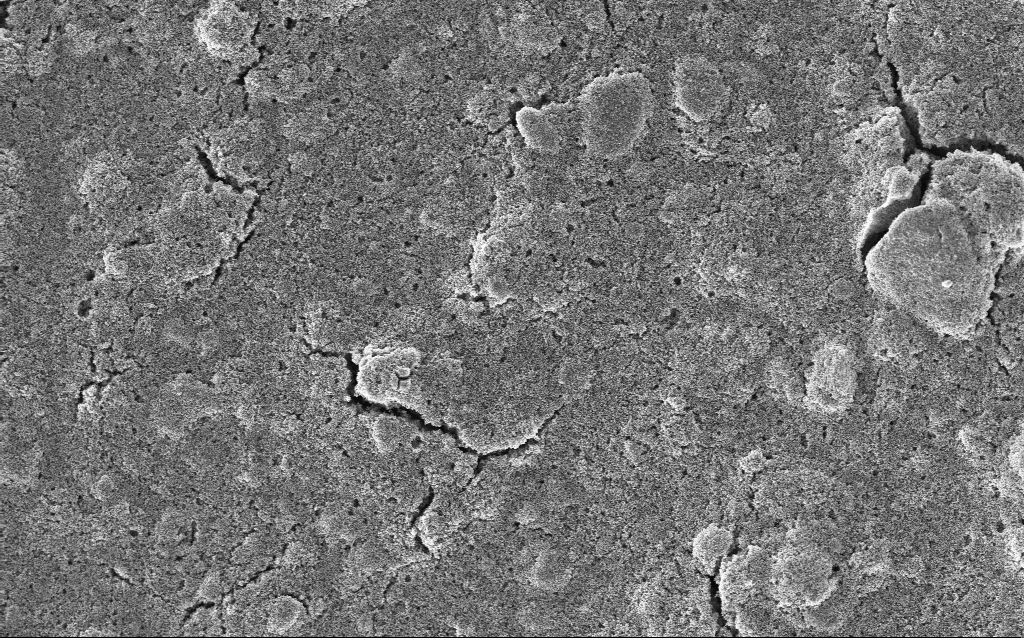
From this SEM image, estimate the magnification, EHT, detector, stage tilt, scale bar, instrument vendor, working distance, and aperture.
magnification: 5 K X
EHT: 5 kV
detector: InLens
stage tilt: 0°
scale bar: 10000 nm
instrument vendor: Zeiss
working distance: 2.9 mm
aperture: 30 µm